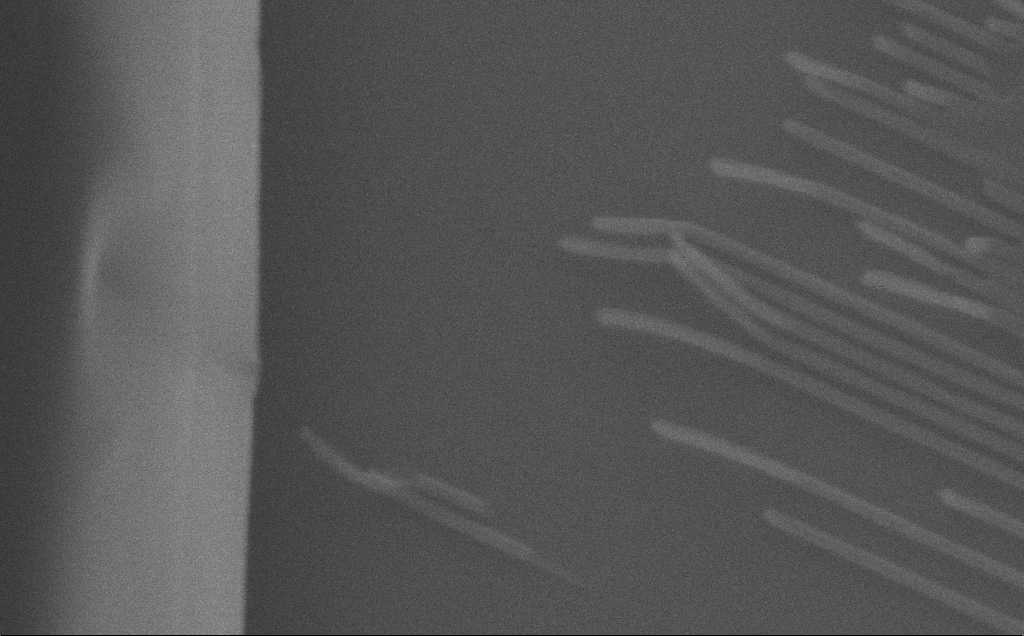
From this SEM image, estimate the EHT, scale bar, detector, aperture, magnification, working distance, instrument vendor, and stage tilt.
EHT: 10 kV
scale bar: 2000 nm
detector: SE2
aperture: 30 µm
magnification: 32.79 K X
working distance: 12 mm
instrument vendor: Zeiss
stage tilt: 0°